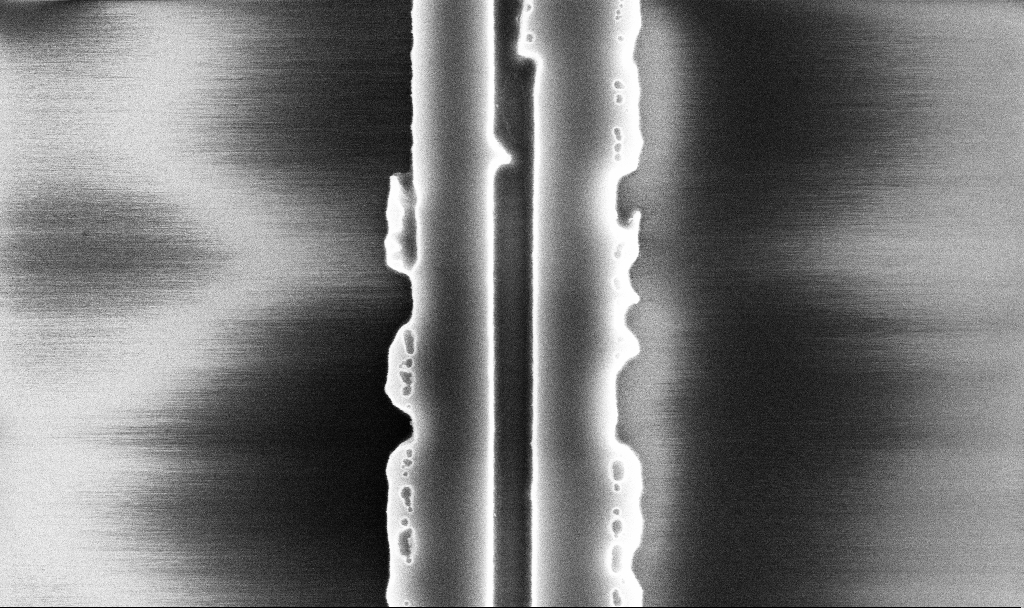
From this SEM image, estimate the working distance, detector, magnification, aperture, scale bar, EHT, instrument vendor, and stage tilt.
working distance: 10.1 mm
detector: InLens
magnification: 62.21 K X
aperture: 30 µm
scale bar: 1000 nm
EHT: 5 kV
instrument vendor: Zeiss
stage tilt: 0°